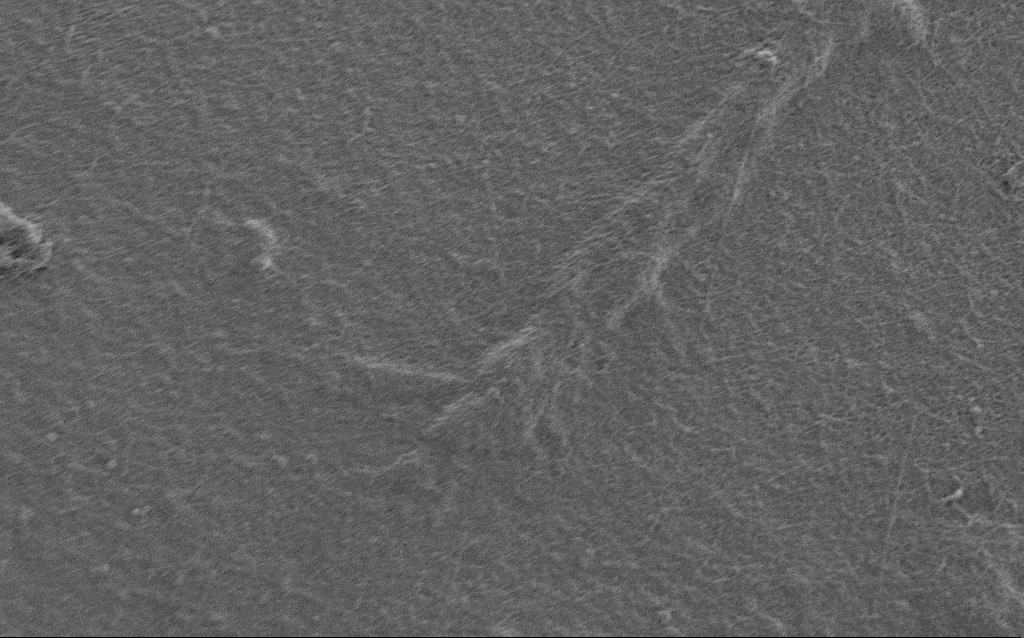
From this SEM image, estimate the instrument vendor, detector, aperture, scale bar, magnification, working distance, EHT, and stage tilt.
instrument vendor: Zeiss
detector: SE2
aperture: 30 µm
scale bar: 2000 nm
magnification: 10 K X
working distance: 6 mm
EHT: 0.9 kV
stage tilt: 0°